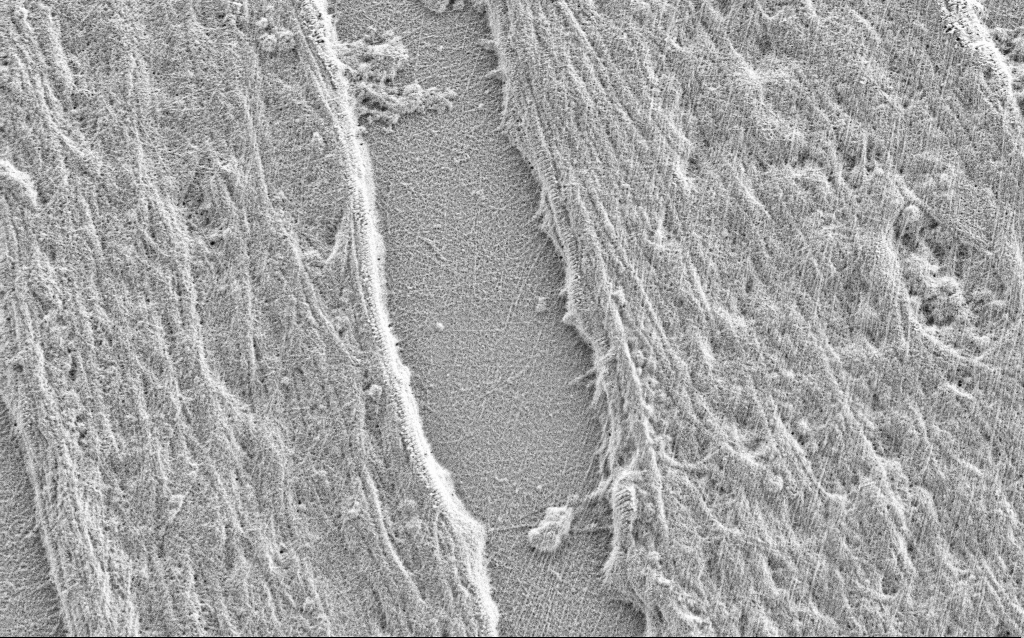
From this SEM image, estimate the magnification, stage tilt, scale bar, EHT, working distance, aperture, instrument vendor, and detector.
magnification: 10 K X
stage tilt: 0°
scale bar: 2000 nm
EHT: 0.9 kV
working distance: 3 mm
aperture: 30 µm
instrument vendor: Zeiss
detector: SE2